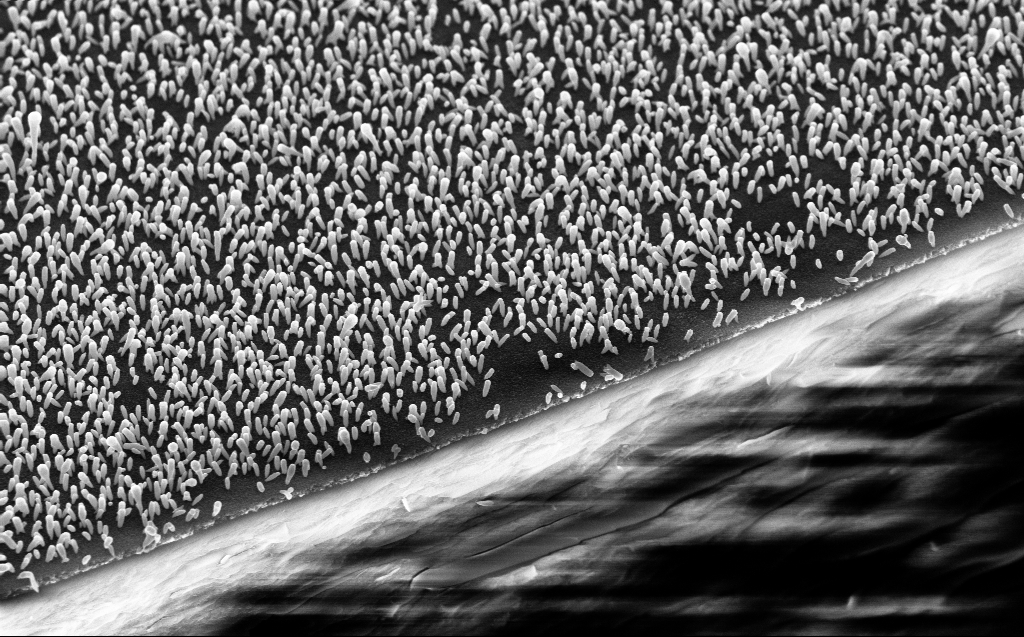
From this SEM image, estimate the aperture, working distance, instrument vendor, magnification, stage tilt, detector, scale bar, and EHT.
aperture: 30 µm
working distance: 5 mm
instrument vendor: Zeiss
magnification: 18.36 K X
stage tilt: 45°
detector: InLens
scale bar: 1000 nm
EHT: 10 kV